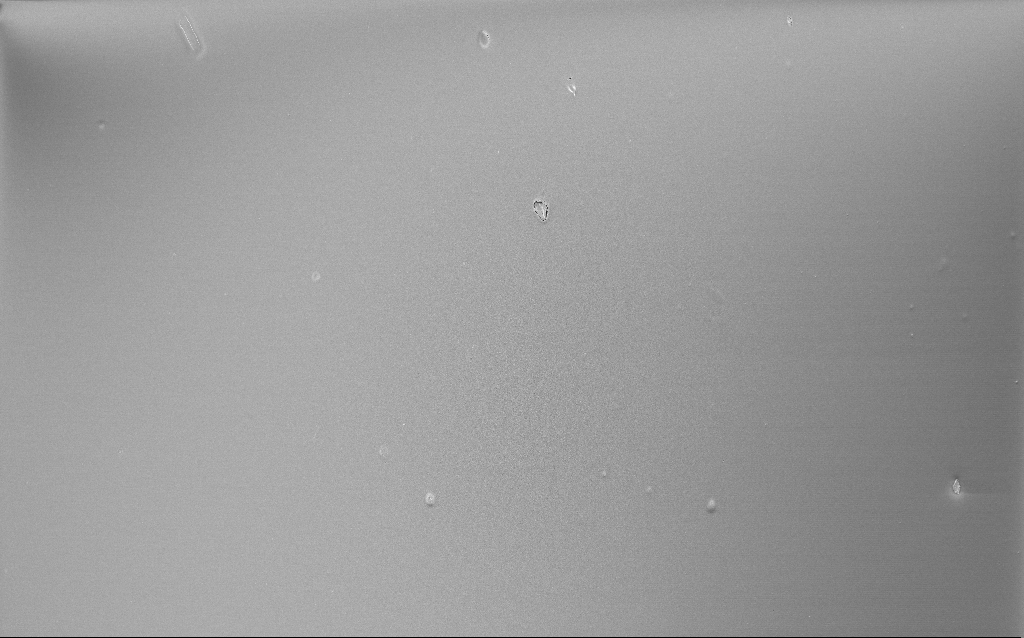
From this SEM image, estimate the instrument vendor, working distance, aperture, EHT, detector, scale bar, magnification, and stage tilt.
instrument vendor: Zeiss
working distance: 2.6 mm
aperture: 30 µm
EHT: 5 kV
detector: InLens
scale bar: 200000 nm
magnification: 0.235 K X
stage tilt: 0°